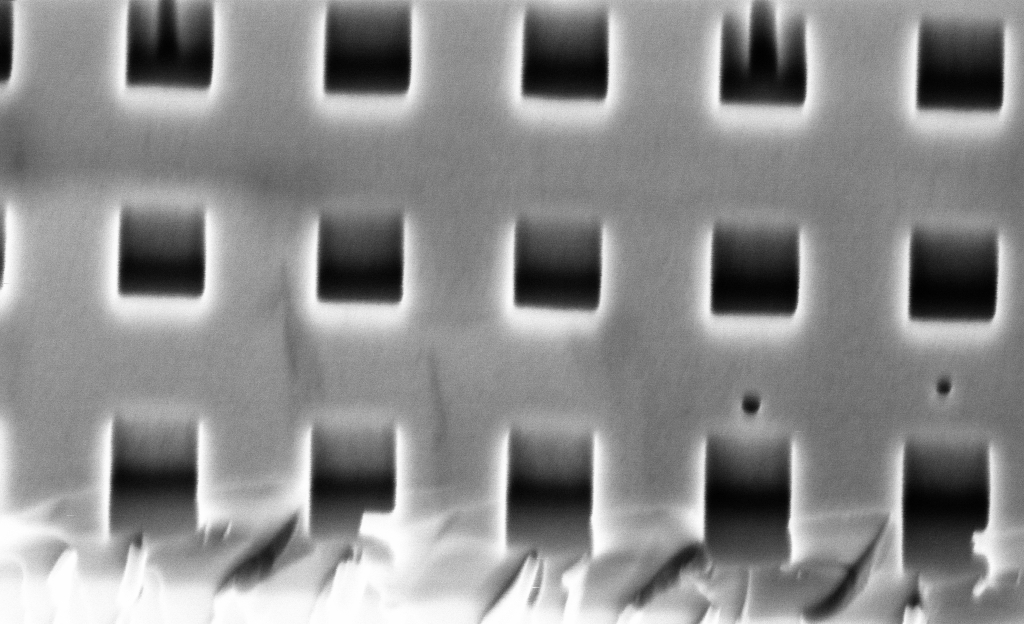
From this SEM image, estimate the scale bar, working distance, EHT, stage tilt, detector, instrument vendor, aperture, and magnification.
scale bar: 200 nm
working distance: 2.7 mm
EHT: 2 kV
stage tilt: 45°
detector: InLens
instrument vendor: Zeiss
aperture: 30 µm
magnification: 146.13 K X